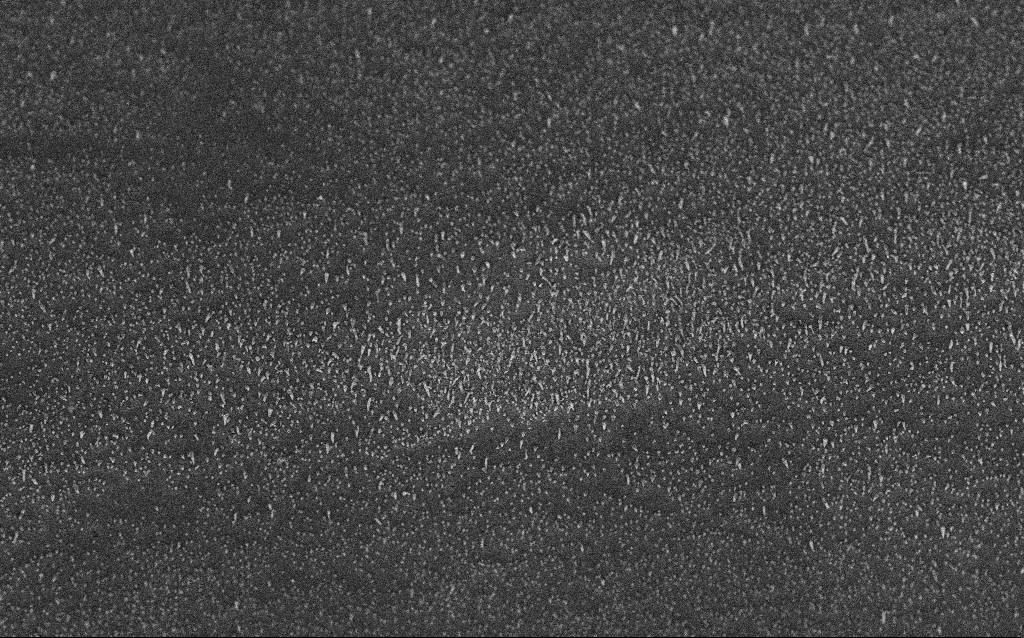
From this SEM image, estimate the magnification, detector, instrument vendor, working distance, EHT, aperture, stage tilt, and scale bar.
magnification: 10 K X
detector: InLens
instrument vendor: Zeiss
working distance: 6.6 mm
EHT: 5 kV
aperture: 30 µm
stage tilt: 45°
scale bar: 2000 nm